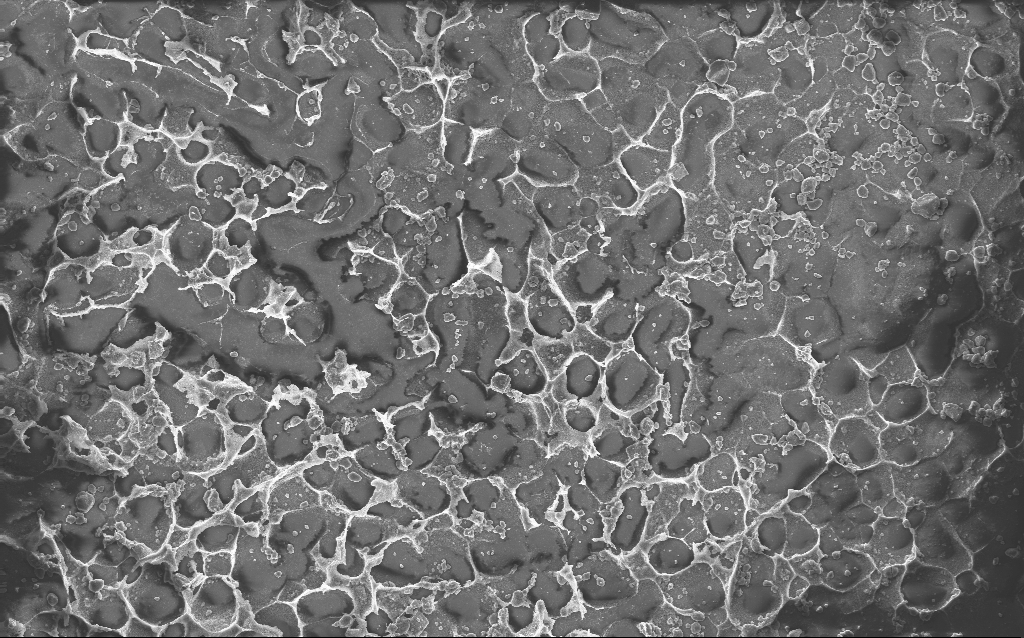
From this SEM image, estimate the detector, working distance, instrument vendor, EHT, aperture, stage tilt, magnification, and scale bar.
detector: InLens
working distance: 2.8 mm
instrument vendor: Zeiss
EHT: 10 kV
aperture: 30 µm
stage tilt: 0°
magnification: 0.792 K X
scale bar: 20000 nm